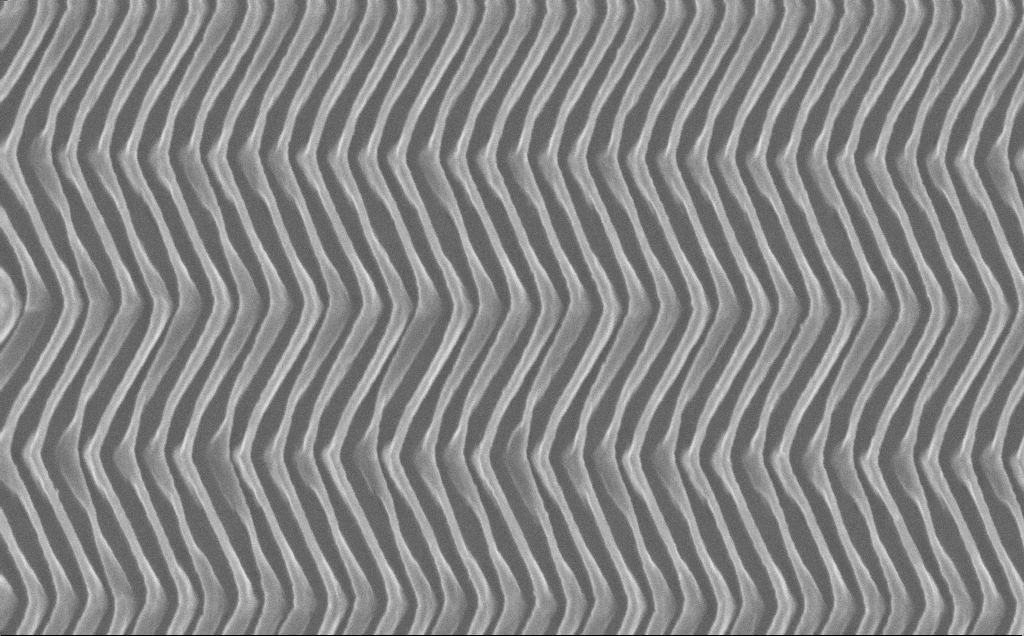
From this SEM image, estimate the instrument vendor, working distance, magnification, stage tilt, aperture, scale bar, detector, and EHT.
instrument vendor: Zeiss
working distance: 7 mm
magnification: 54.99 K X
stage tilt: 0°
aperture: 30 µm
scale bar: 1000 nm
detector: InLens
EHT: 10 kV